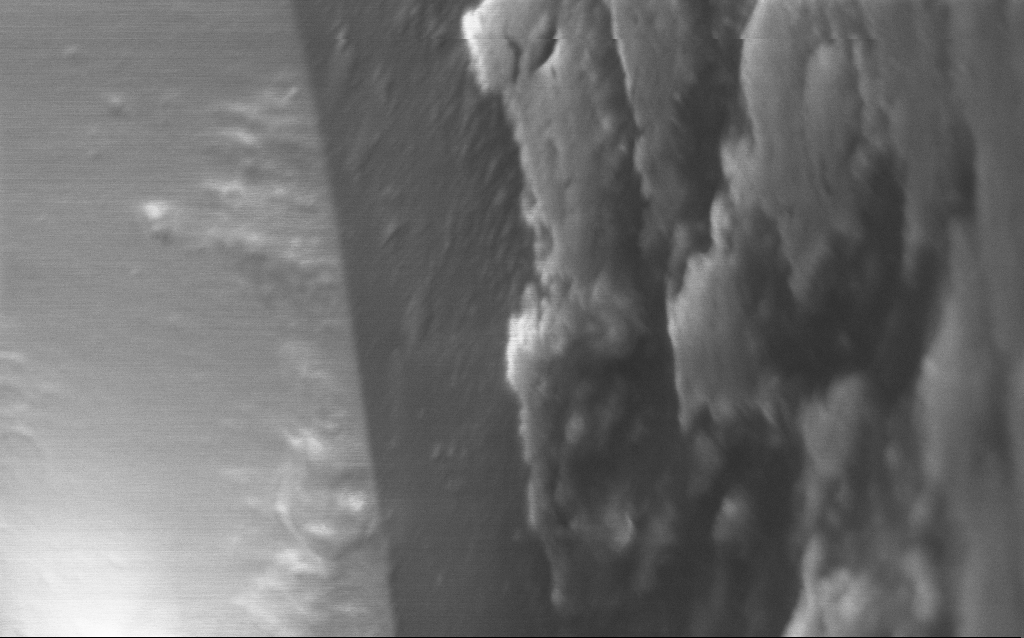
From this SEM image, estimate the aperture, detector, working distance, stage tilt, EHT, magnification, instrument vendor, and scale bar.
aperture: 30 µm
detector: InLens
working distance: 5 mm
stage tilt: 45°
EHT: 1 kV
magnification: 77.65 K X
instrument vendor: Zeiss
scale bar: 200 nm